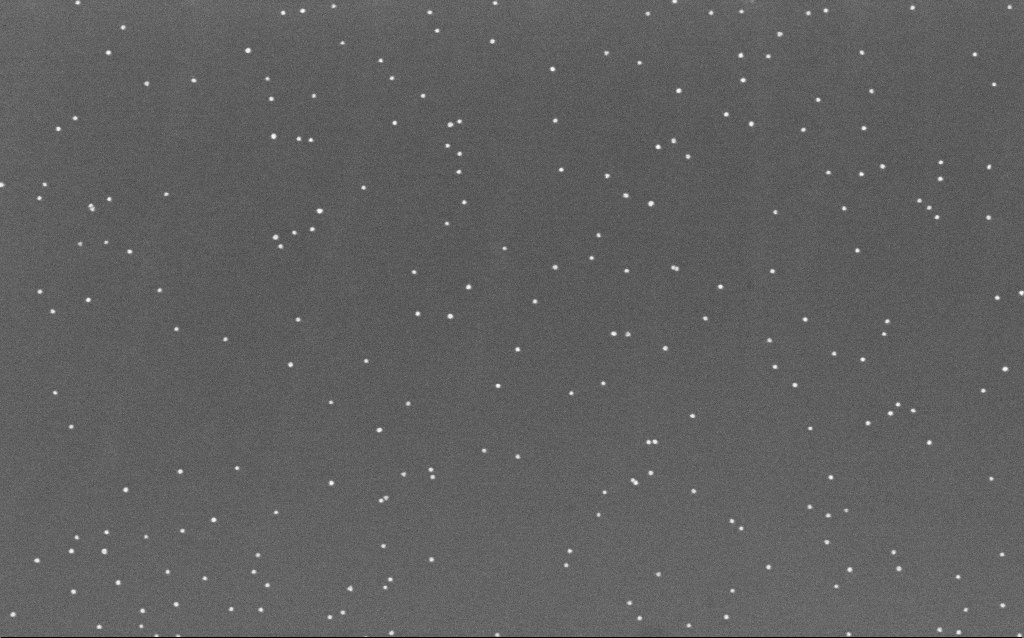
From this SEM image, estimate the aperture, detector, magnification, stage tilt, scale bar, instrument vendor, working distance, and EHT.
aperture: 30 µm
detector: InLens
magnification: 100 K X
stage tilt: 0°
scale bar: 200 nm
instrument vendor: Zeiss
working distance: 6.6 mm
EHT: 10 kV